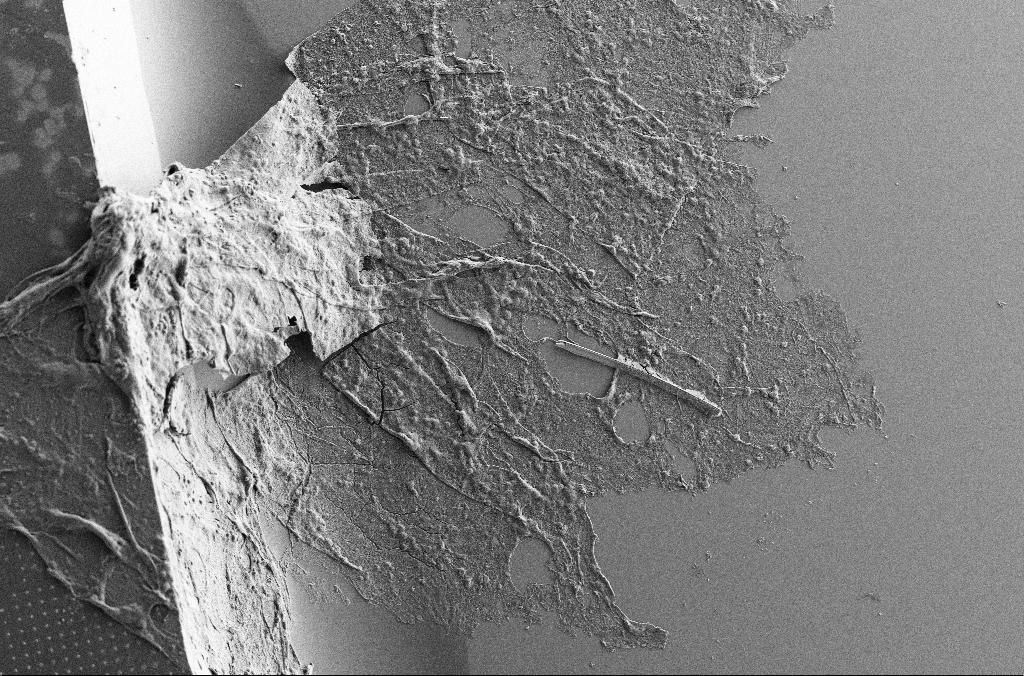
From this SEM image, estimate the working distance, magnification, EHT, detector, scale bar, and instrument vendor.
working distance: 3.7 mm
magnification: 0.15 K X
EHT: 7 kV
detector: SE2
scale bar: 100000 nm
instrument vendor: Zeiss